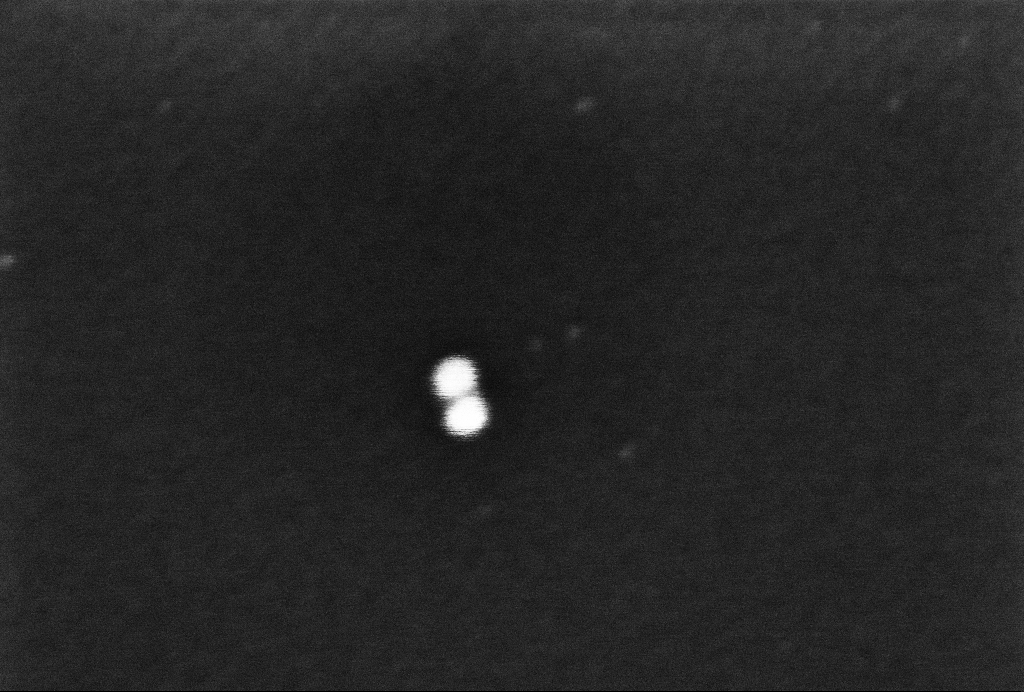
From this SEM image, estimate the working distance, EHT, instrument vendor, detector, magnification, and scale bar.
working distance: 3.3 mm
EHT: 2 kV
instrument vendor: Zeiss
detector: InLens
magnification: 574.46 K X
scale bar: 100 nm